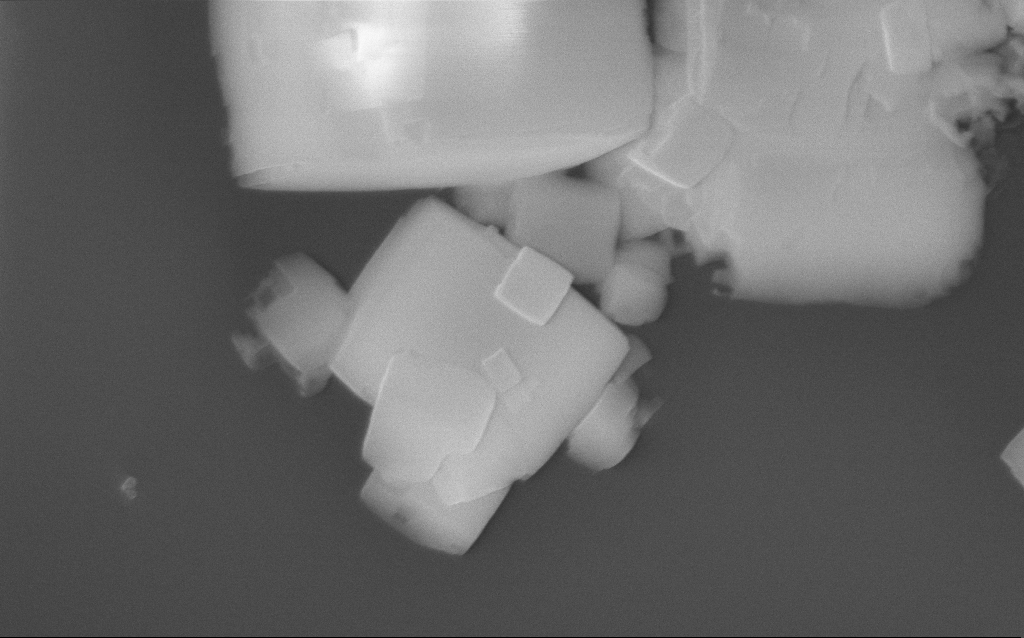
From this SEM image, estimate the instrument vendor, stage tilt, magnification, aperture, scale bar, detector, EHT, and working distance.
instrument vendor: Zeiss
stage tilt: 0°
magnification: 65.8 K X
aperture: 30 µm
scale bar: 1000 nm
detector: InLens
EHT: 10 kV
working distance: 2 mm